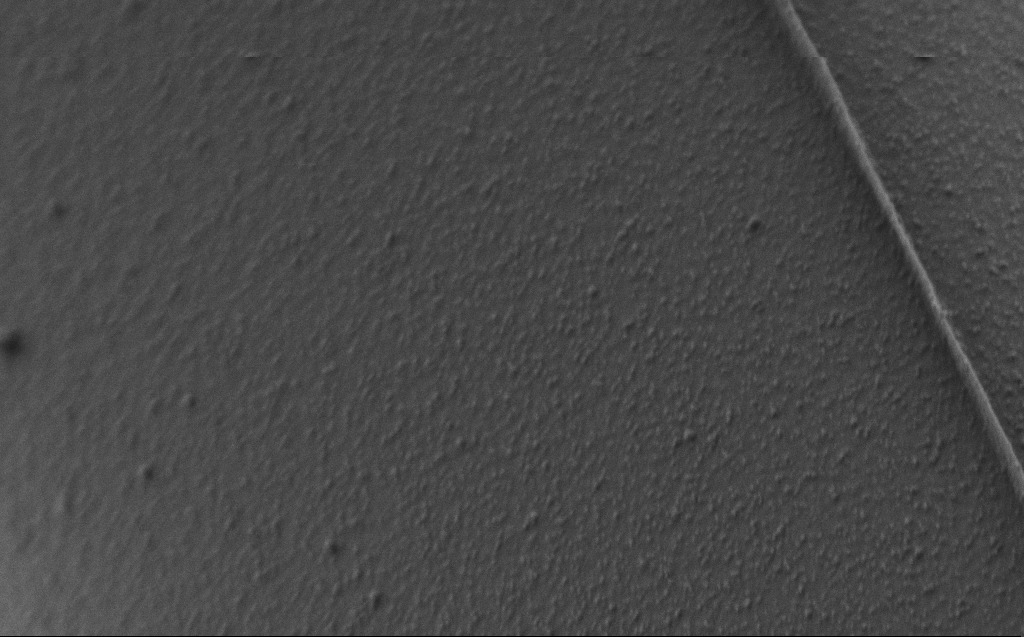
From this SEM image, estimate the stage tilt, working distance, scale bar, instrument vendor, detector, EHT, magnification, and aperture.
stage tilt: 45°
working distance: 4 mm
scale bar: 1000 nm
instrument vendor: Zeiss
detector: SE2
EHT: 0.8 kV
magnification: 50 K X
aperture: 30 µm